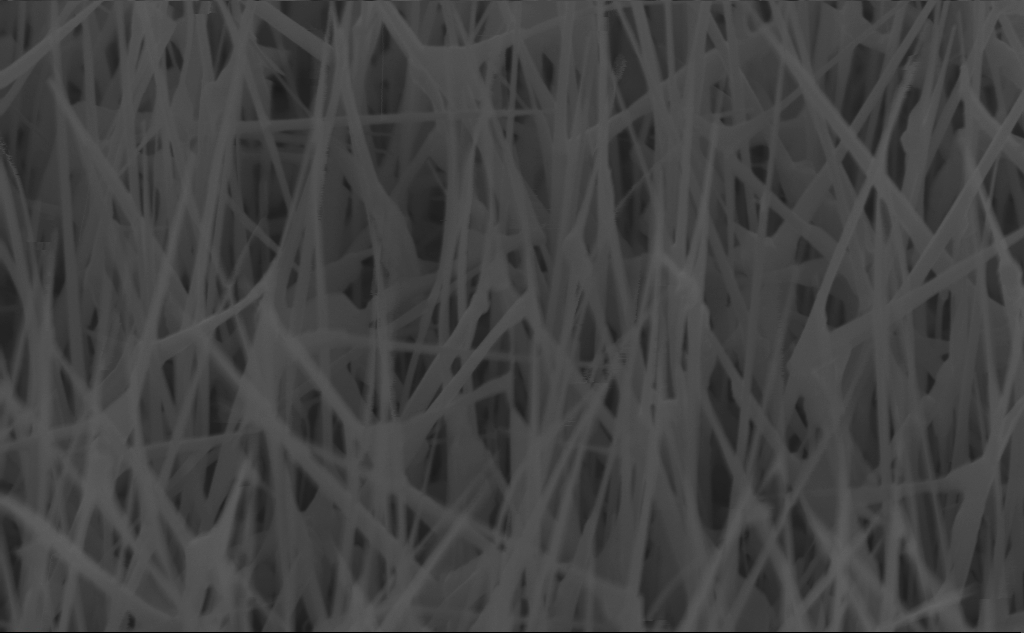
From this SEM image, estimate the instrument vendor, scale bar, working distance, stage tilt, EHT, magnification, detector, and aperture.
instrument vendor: Zeiss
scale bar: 200 nm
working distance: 4 mm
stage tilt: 45°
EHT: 10 kV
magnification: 80.54 K X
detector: InLens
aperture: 30 µm